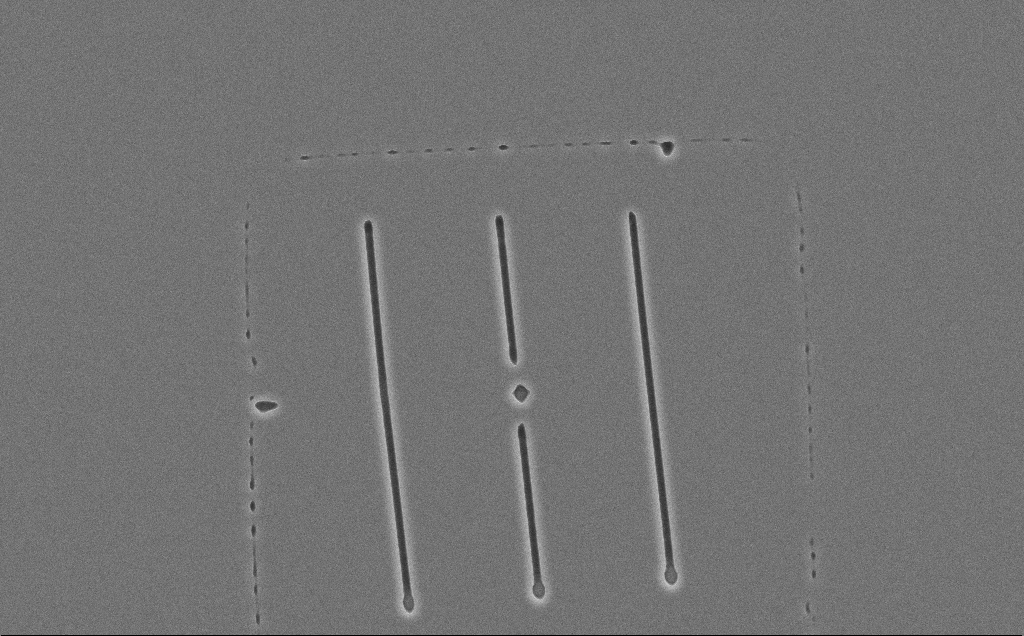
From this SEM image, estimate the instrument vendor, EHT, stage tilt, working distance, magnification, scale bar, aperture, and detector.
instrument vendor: Zeiss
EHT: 10 kV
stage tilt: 0°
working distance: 12 mm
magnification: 2.41 K X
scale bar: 20000 nm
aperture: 30 µm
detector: SE2